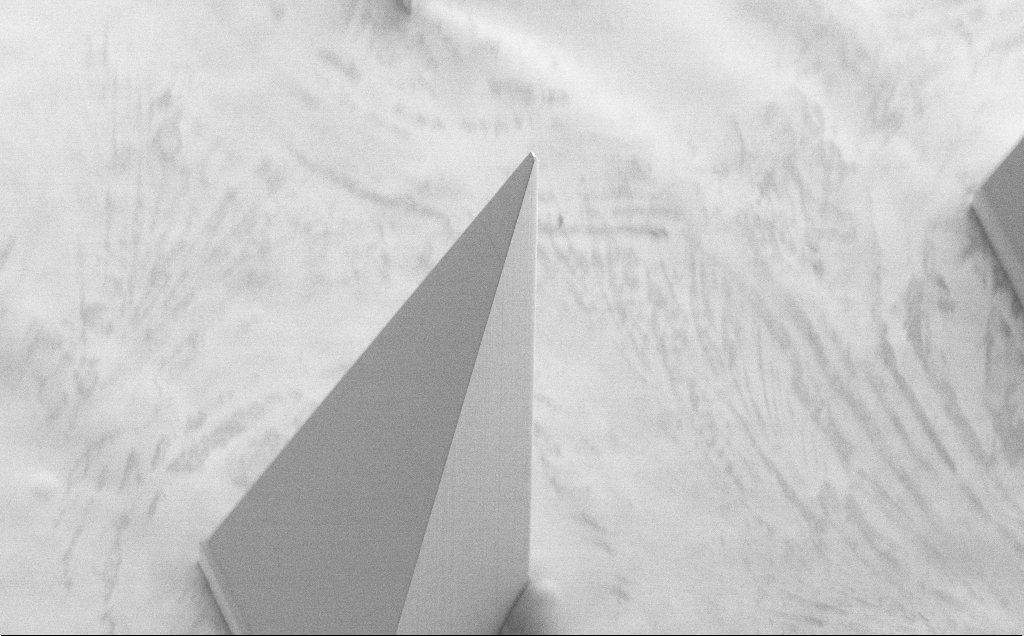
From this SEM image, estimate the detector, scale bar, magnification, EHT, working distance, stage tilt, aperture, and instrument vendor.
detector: SE2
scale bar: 100000 nm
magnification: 0.204 K X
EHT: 5 kV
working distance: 8 mm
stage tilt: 40°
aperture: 30 µm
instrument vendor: Zeiss